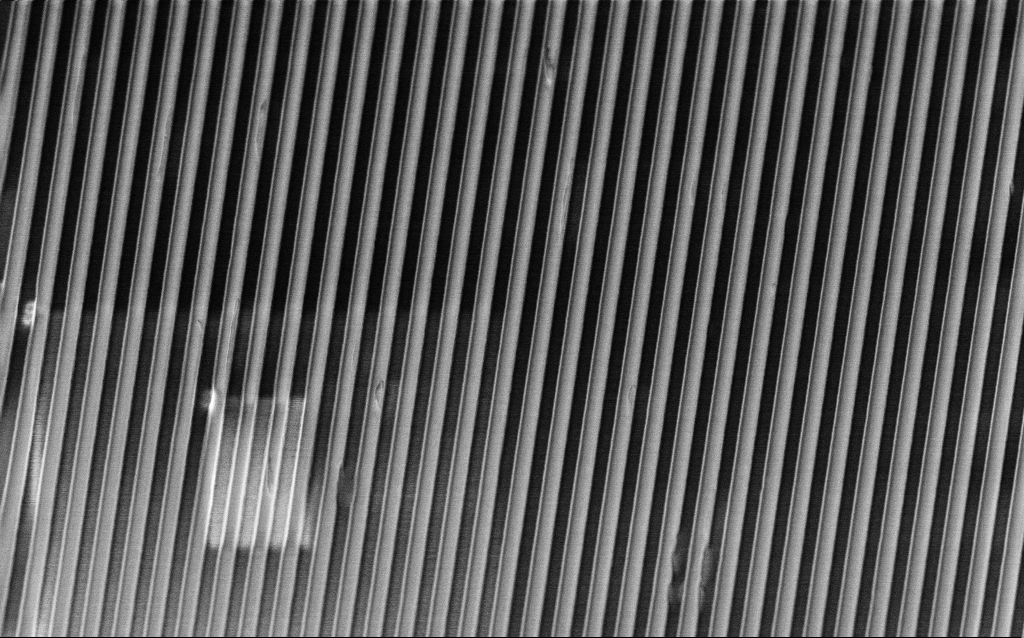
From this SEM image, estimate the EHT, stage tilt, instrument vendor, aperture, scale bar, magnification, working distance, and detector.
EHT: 2 kV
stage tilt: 45°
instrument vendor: Zeiss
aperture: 30 µm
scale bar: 1000 nm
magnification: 23.04 K X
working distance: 4.1 mm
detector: InLens